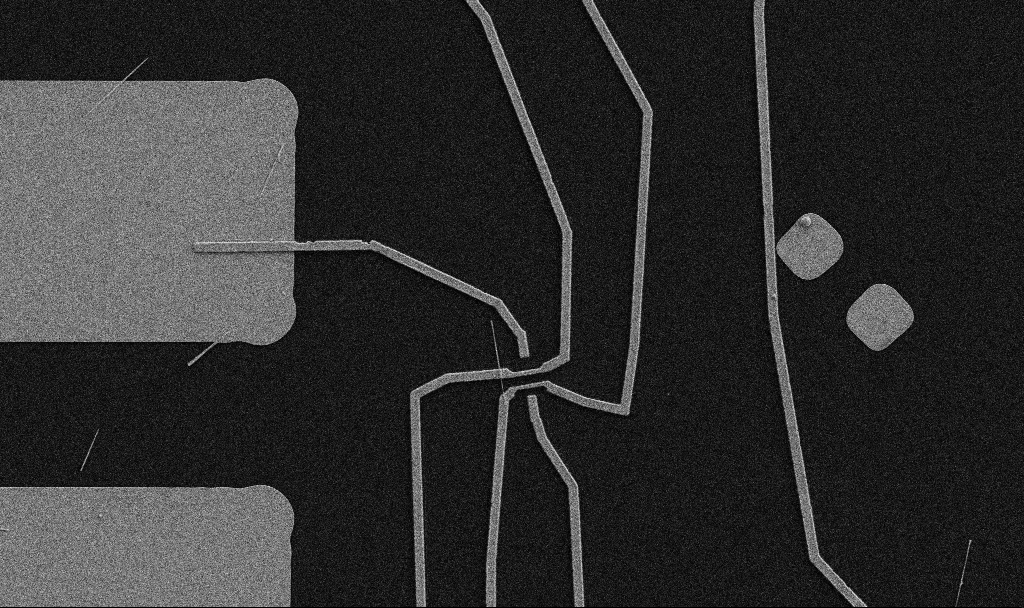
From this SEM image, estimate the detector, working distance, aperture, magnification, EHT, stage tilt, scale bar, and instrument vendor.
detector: SE2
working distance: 10.7 mm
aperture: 30 µm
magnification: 5 K X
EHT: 5 kV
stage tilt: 0°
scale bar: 10000 nm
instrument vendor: Zeiss